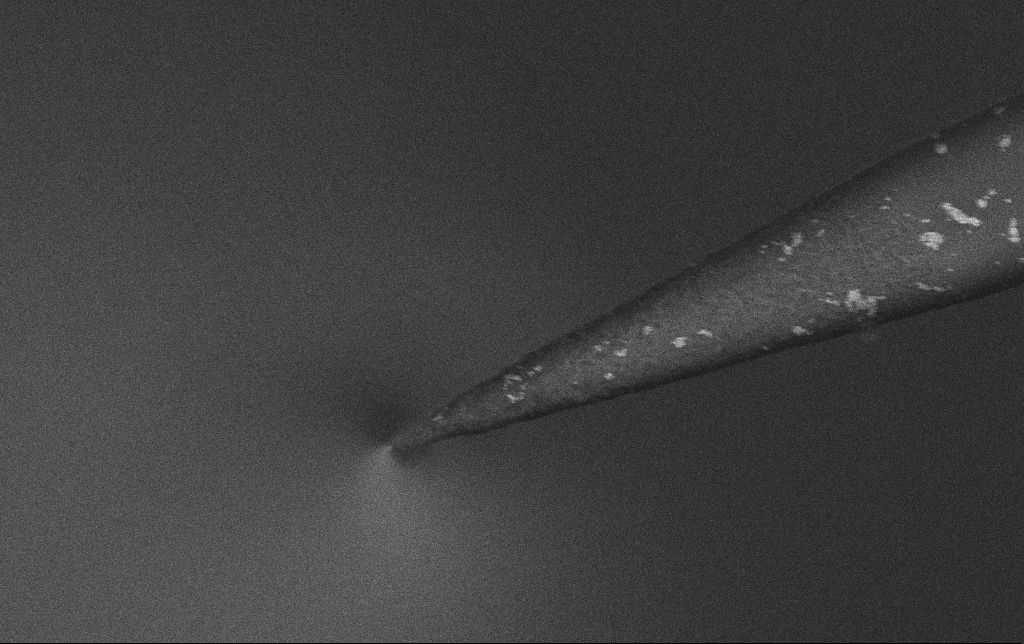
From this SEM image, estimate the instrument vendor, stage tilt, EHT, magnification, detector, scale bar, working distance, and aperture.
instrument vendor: Zeiss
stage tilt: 0°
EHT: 3 kV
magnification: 40 K X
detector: InLens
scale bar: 1000 nm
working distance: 6.6 mm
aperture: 30 µm